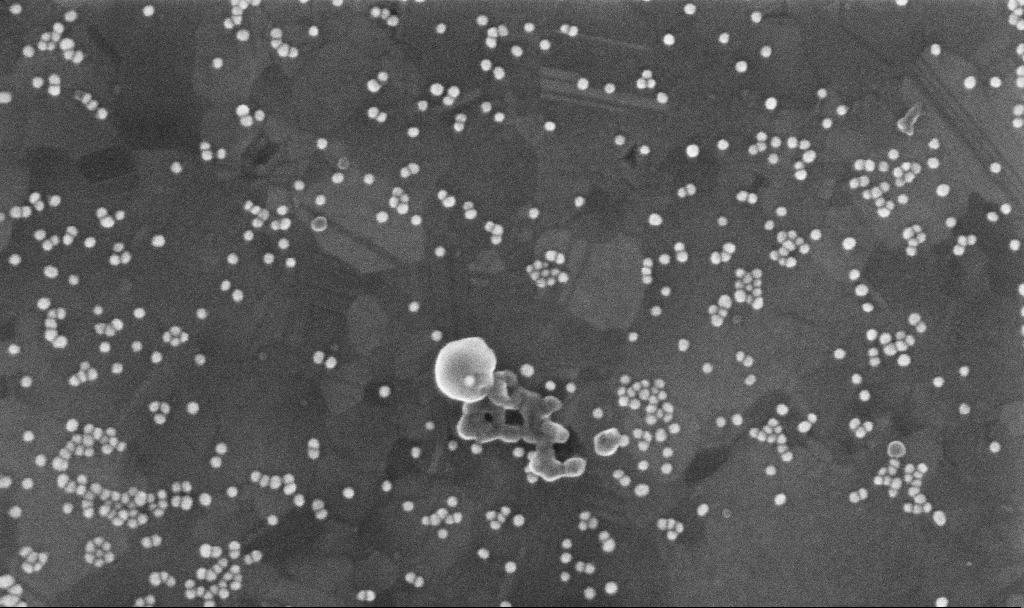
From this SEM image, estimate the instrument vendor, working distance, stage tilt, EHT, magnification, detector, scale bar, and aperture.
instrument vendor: Zeiss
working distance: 3.7 mm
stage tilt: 0°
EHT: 10 kV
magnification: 200 K X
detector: InLens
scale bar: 200 nm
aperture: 30 µm